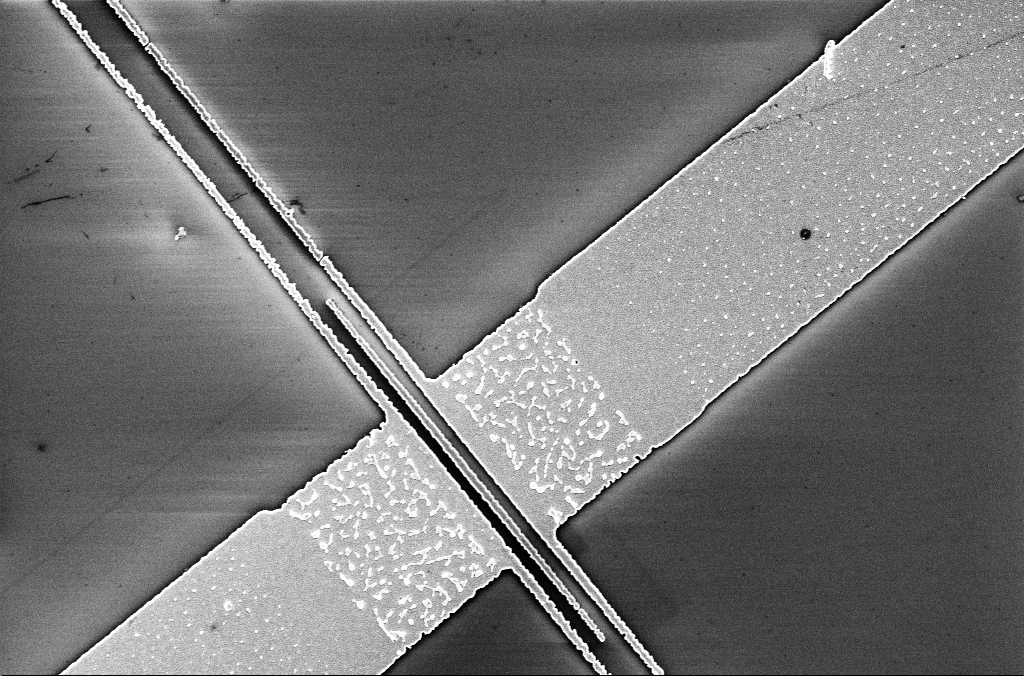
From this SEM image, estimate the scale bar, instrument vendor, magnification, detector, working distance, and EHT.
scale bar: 2000 nm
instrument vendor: Zeiss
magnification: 14.56 K X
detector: InLens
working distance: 3.3 mm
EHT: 5 kV